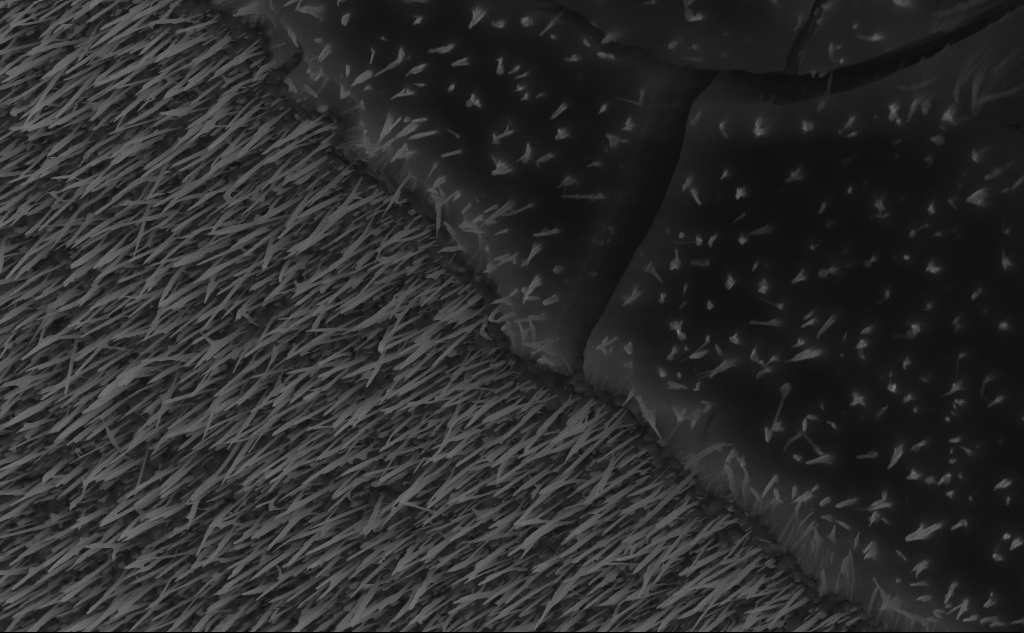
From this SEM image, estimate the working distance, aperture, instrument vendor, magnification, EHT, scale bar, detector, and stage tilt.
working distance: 6 mm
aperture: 30 µm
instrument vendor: Zeiss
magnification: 23.9 K X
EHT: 10 kV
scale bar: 2000 nm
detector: InLens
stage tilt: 45°